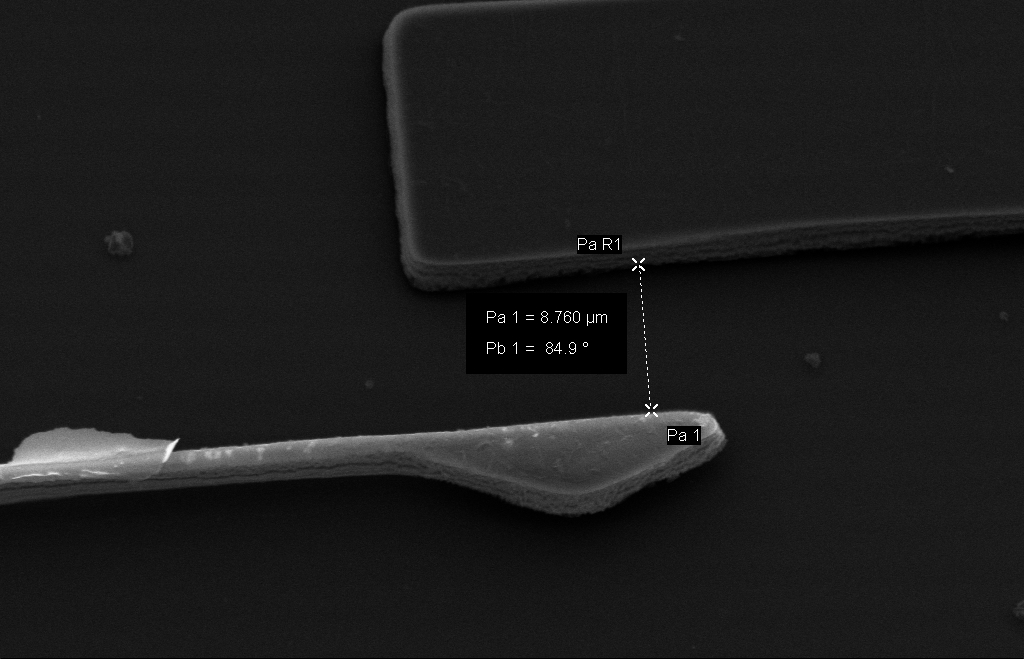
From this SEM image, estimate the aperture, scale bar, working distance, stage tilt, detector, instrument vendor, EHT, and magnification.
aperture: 30 µm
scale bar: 2000 nm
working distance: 20 mm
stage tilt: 23.3°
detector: SE2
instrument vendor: Zeiss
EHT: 10 kV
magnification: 6.14 K X